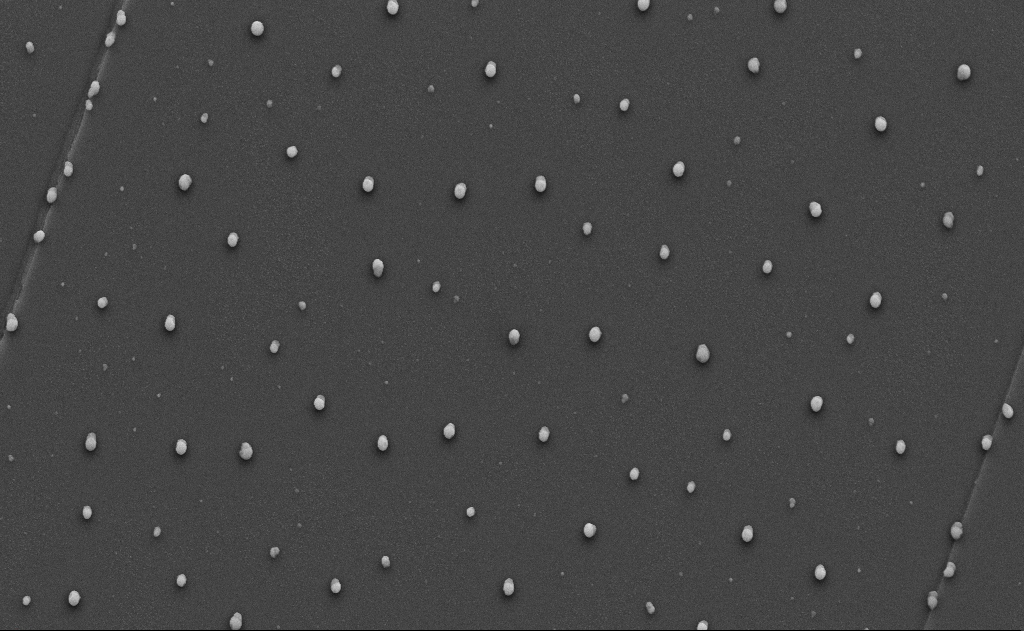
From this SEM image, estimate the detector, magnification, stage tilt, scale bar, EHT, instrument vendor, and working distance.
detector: SE2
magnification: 10 K X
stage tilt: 0°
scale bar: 2000 nm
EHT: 10 kV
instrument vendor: Zeiss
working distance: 12 mm